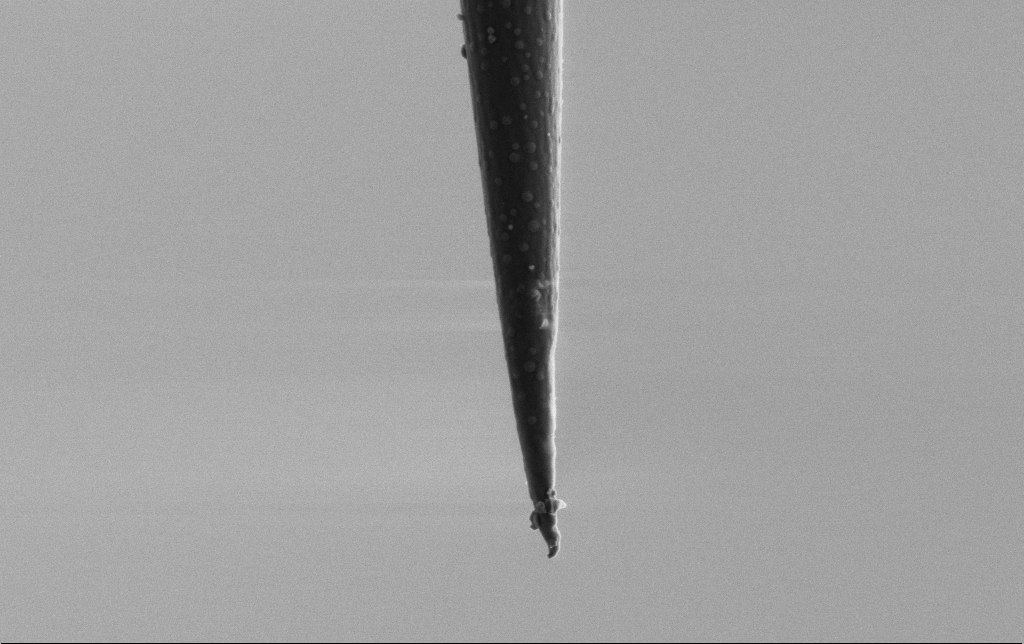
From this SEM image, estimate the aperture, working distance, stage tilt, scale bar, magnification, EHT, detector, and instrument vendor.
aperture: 30 µm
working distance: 6.5 mm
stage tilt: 0°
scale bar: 1000 nm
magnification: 25 K X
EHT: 2 kV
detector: SE2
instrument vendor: Zeiss